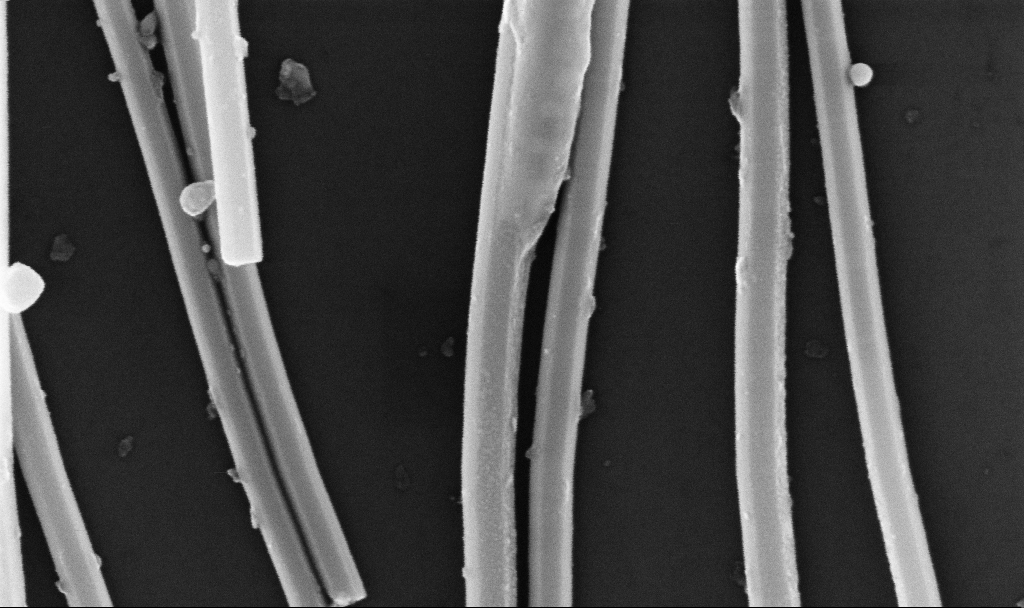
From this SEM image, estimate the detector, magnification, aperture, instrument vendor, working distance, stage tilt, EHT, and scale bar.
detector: InLens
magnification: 155.83 K X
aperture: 30 µm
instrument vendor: Zeiss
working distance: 6.7 mm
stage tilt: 0°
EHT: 10 kV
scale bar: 200 nm